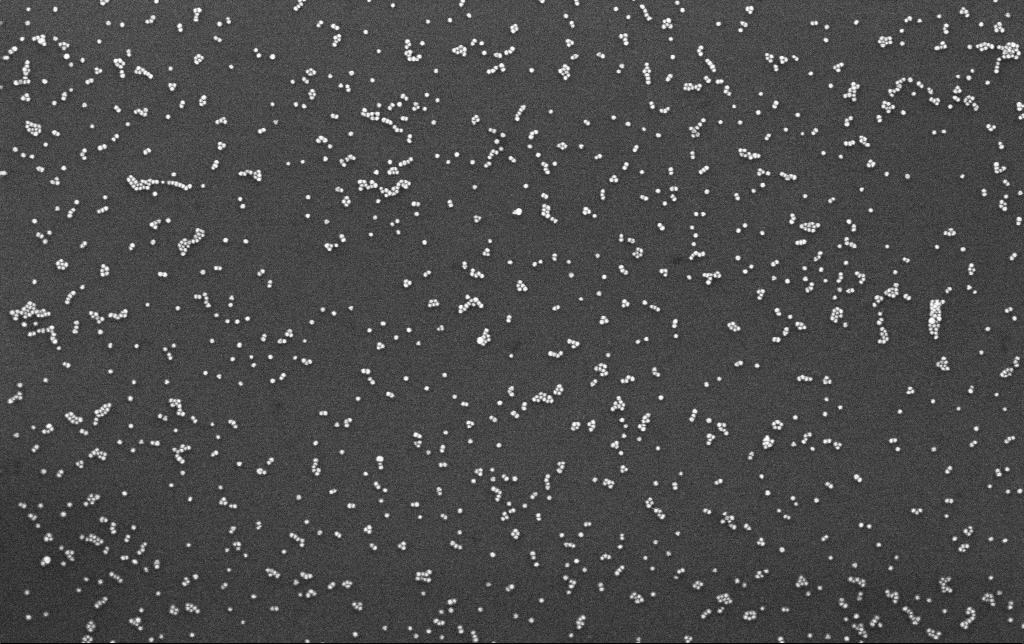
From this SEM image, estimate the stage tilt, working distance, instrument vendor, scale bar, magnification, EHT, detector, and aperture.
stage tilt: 0°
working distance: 3.1 mm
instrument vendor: Zeiss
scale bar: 200 nm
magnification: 100 K X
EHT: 10 kV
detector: InLens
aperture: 30 µm